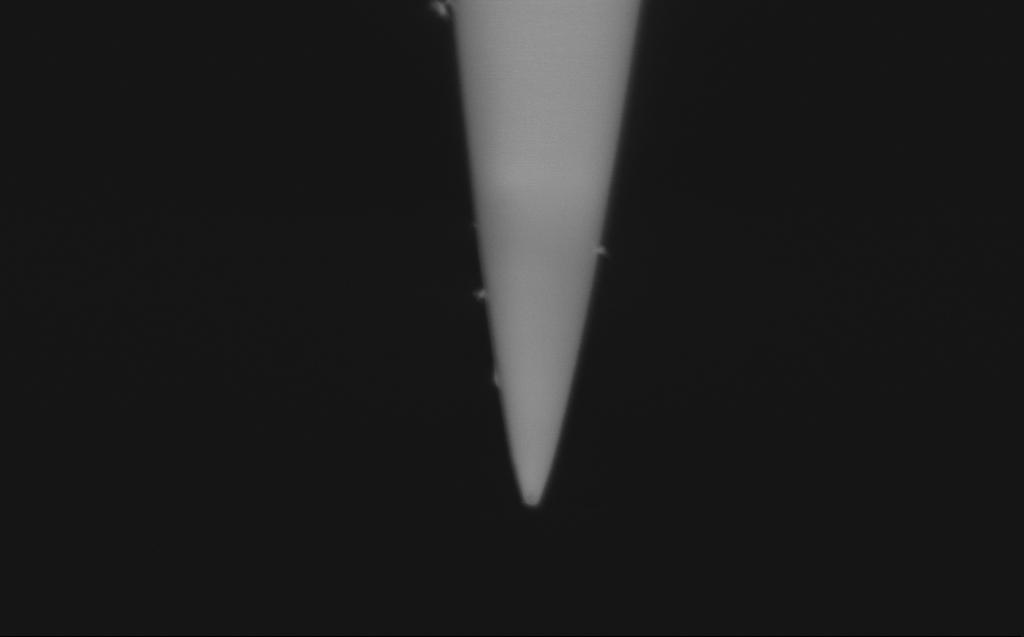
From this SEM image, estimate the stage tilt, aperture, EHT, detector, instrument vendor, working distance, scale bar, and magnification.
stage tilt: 45.1°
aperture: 30 µm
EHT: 0.75 kV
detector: InLens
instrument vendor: Zeiss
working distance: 3 mm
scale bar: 1000 nm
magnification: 63.38 K X